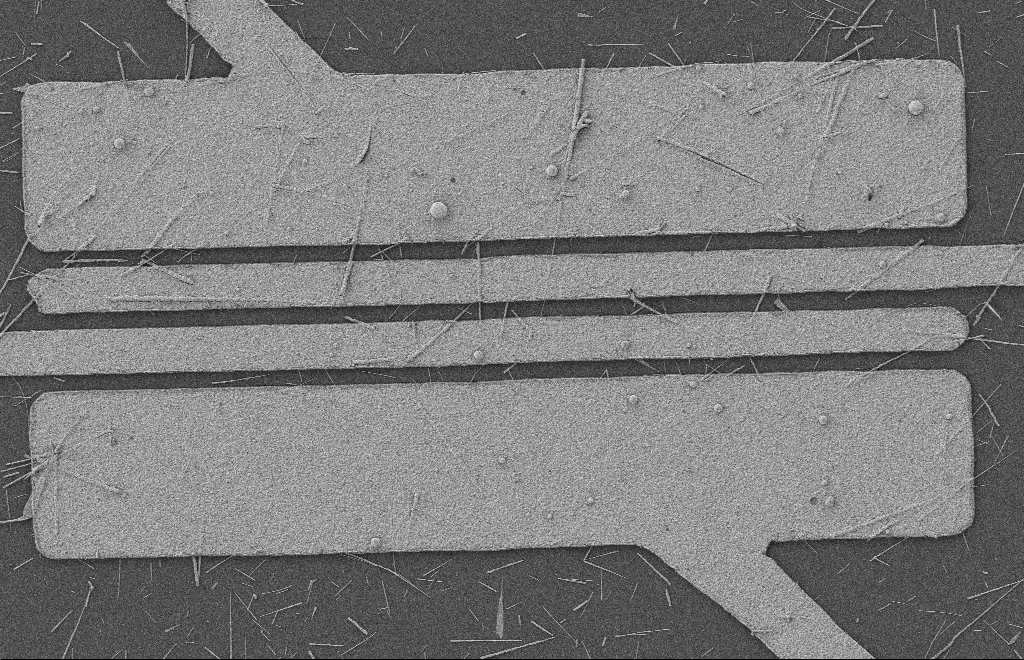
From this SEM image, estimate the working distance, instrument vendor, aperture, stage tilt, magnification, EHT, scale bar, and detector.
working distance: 8 mm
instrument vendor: Zeiss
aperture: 20 µm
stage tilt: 0°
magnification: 5.65 K X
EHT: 2 kV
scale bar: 2000 nm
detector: SE2